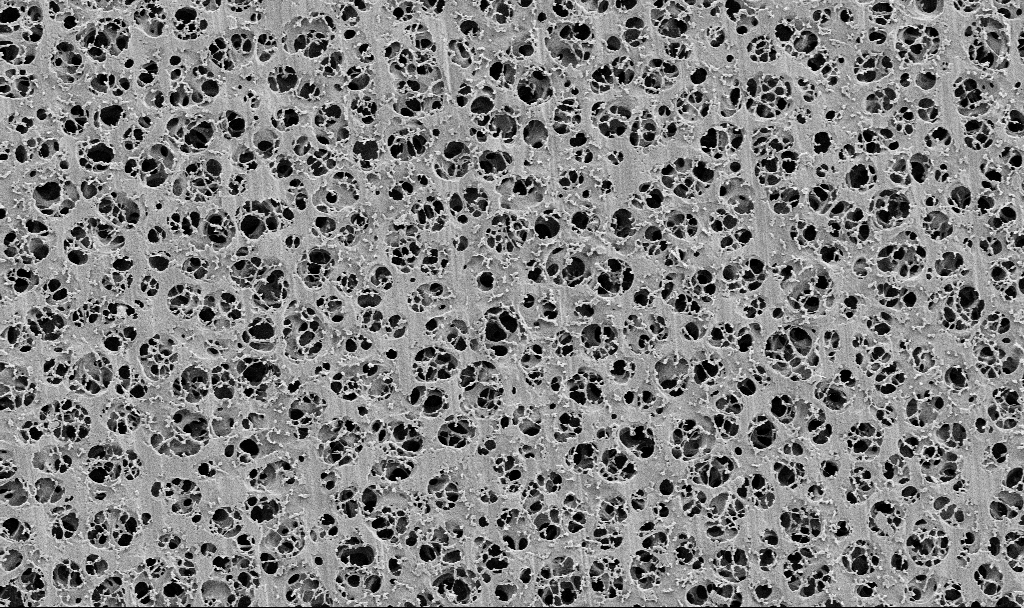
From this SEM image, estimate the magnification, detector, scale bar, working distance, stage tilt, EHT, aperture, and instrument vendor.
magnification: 5 K X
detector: SE2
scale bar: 10000 nm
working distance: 3.7 mm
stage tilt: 0°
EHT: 2 kV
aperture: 30 µm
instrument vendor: Zeiss